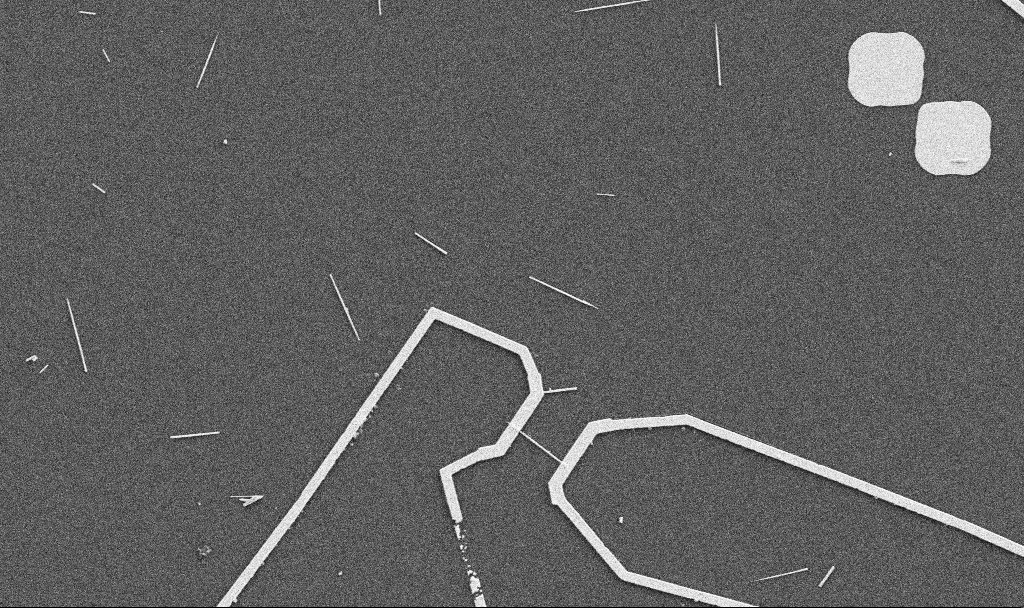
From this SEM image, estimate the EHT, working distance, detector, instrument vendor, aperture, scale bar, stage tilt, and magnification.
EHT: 5 kV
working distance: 10.7 mm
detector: SE2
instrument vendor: Zeiss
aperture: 30 µm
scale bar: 10000 nm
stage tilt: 0°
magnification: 5 K X